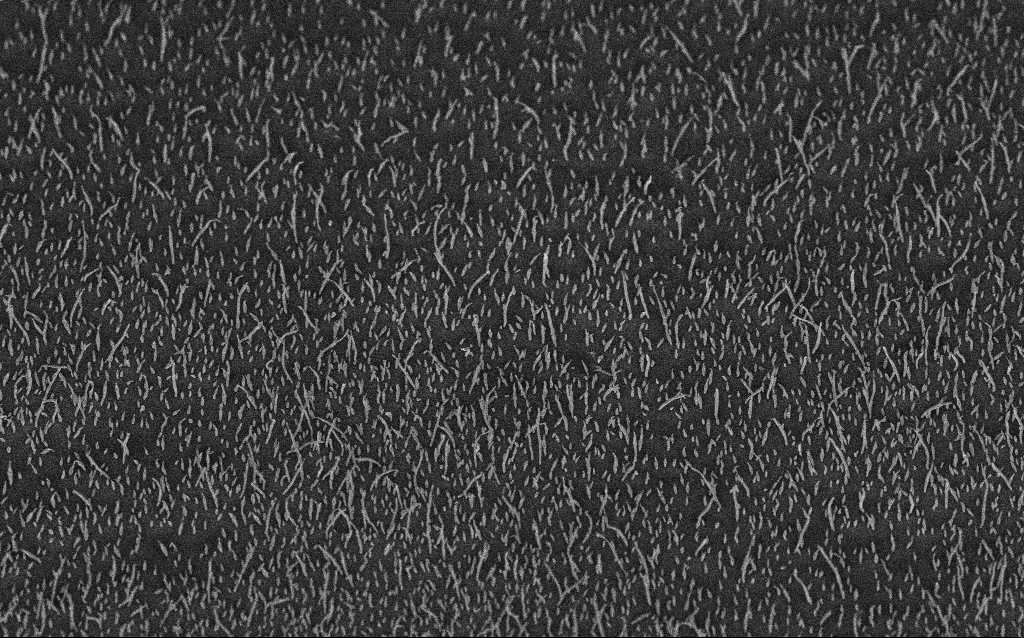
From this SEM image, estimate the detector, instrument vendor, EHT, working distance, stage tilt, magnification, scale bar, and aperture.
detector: InLens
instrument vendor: Zeiss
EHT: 5 kV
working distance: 7 mm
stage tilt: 45°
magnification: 10 K X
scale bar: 2000 nm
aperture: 30 µm